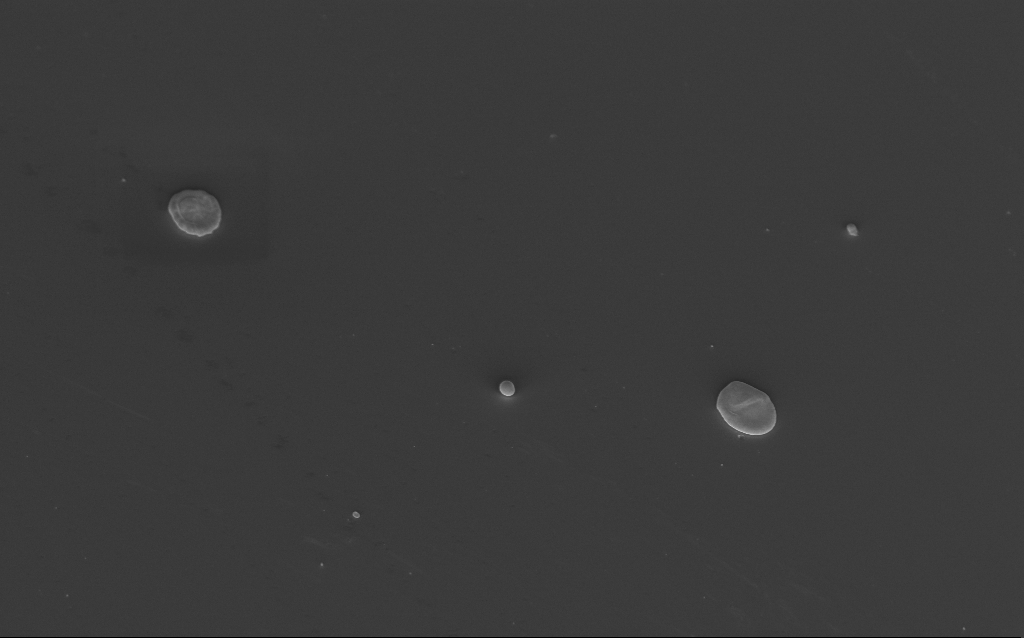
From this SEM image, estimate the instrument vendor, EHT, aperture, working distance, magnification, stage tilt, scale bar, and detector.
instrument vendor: Zeiss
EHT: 3 kV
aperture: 30 µm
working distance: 5 mm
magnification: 9.66 K X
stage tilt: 40°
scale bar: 2000 nm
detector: InLens